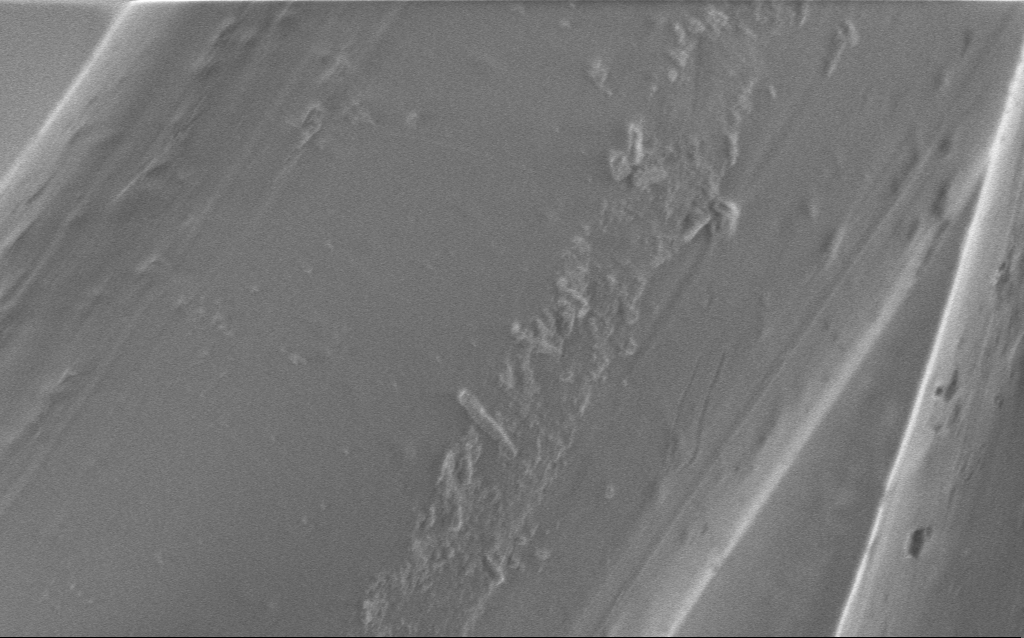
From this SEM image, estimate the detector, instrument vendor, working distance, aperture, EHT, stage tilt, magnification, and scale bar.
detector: InLens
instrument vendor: Zeiss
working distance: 4 mm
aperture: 30 µm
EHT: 1 kV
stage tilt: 0°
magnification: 12.48 K X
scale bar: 1000 nm